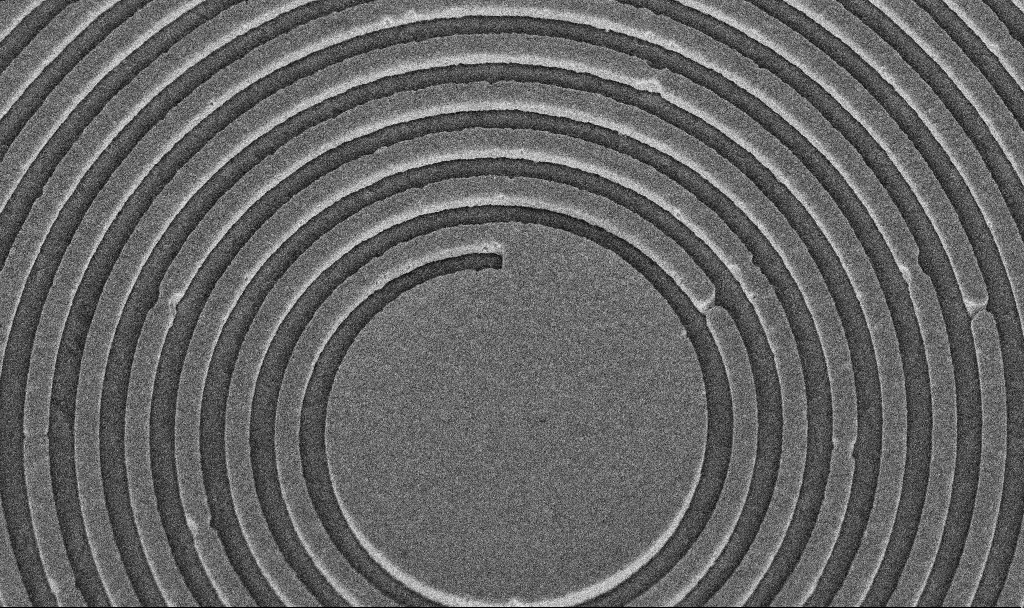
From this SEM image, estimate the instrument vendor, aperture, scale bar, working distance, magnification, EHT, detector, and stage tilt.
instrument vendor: Zeiss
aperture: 30 µm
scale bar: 2000 nm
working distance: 8.7 mm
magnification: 28.65 K X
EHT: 5 kV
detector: InLens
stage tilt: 45°